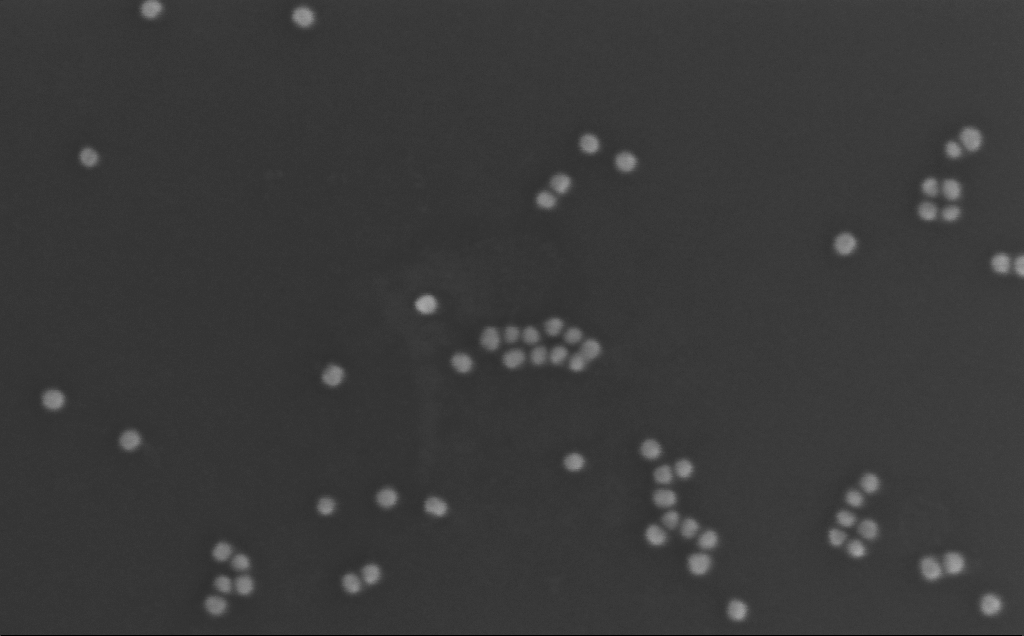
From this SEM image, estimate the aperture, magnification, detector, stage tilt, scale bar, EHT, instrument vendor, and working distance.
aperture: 30 µm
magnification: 446.2 K X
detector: InLens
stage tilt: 0°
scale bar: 100 nm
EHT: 10 kV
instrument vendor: Zeiss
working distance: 3 mm